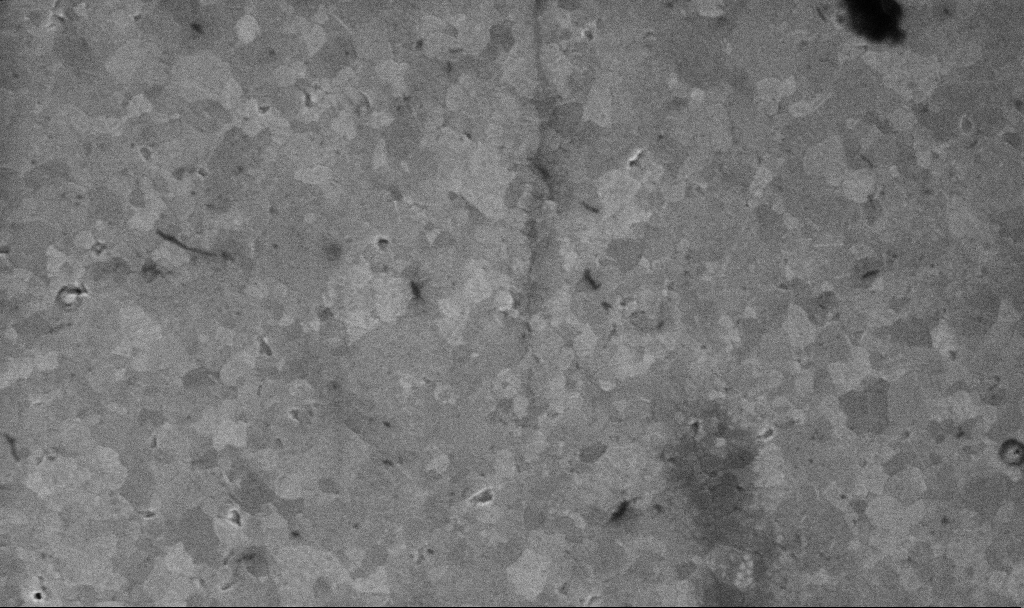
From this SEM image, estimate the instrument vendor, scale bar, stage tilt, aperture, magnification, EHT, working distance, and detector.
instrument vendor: Zeiss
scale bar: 1000 nm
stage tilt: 0°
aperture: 30 µm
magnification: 50.56 K X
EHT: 10 kV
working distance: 3.1 mm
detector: InLens